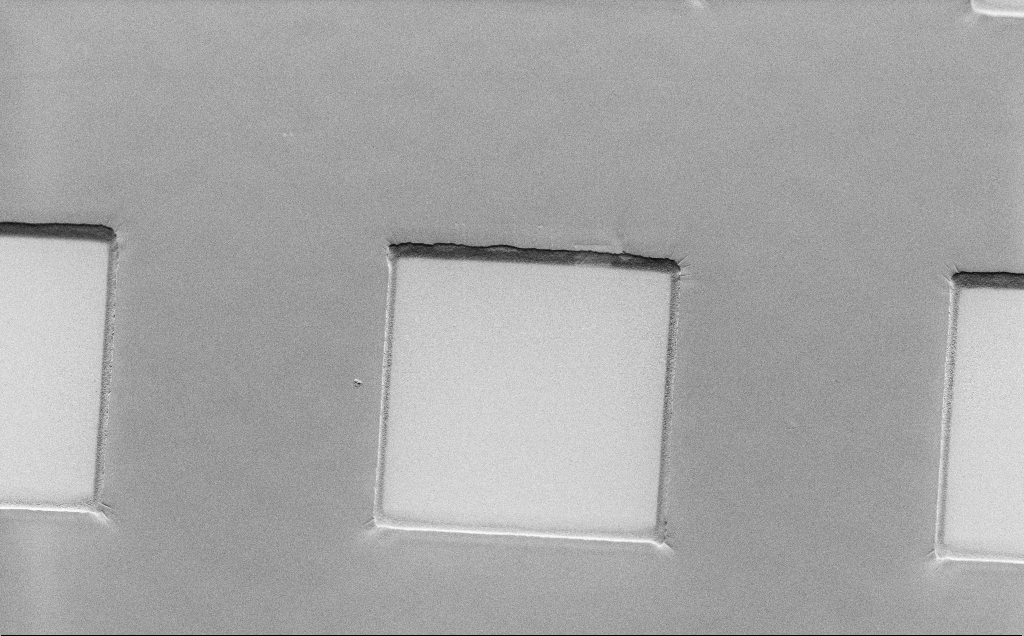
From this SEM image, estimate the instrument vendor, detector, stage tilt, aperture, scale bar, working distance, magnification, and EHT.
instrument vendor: Zeiss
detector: SE2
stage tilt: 45°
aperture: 30 µm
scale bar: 20000 nm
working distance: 7 mm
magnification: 2.06 K X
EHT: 1.5 kV